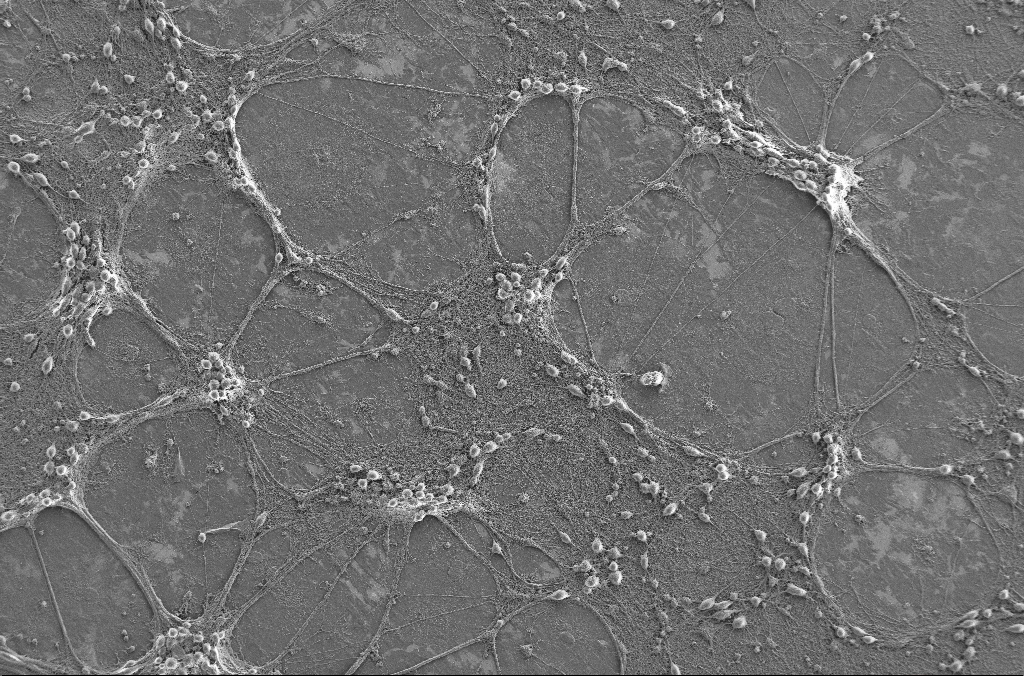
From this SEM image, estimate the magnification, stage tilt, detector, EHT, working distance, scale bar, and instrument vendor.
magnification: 0.5 K X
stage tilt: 0°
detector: SE2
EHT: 2 kV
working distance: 4.1 mm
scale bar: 100000 nm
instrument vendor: Zeiss